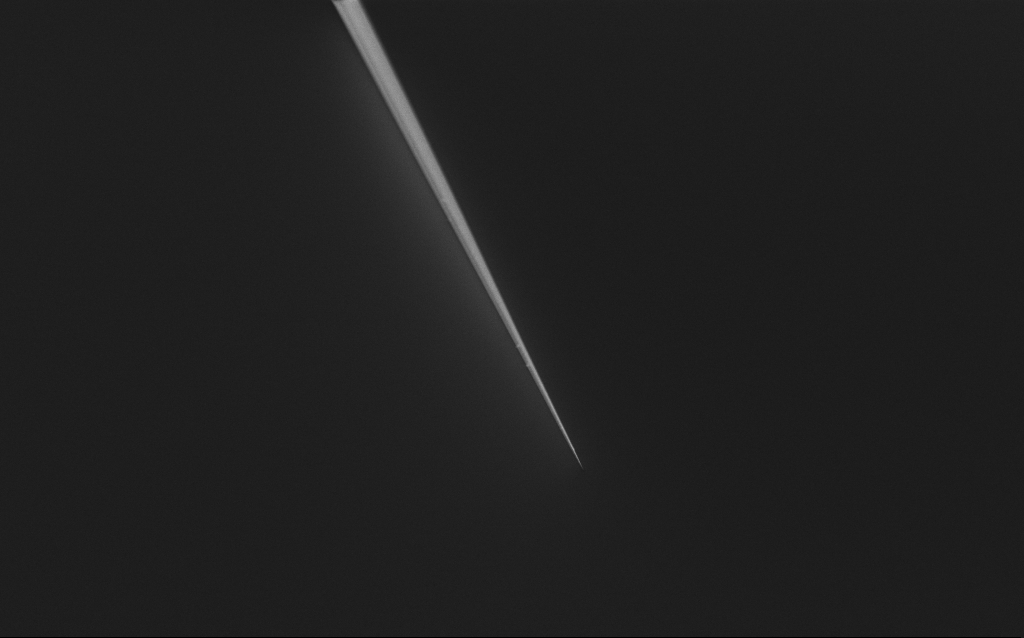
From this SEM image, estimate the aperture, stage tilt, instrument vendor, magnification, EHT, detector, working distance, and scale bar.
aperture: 30 µm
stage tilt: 45°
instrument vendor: Zeiss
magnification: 1 K X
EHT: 1 kV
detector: InLens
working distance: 7 mm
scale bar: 20000 nm